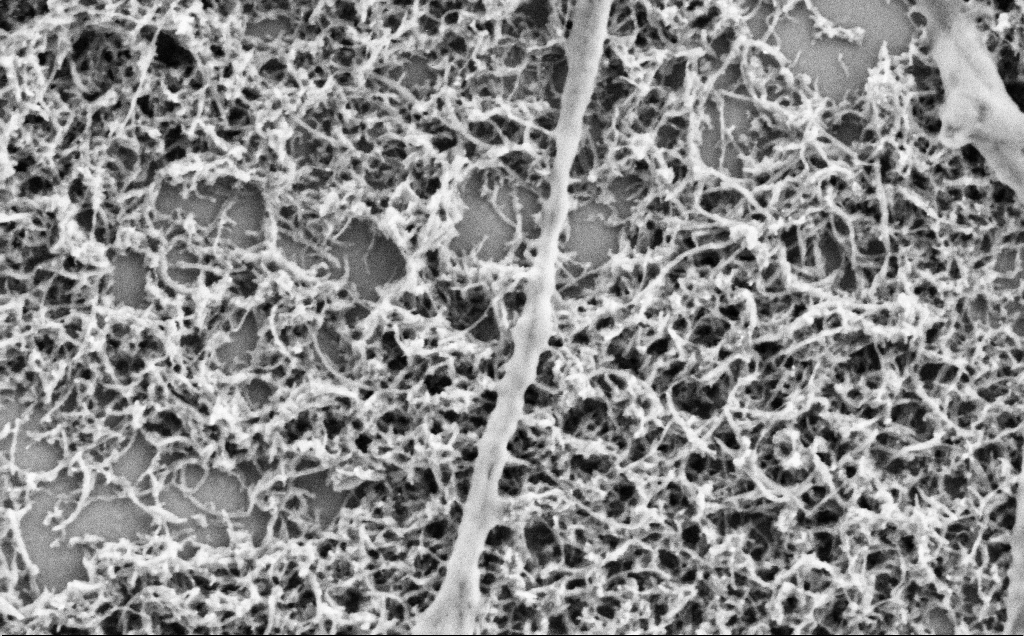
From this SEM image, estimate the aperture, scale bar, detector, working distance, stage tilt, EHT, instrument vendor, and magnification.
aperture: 30 µm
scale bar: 1000 nm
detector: SE2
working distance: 7.1 mm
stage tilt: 0°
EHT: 2 kV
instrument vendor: Zeiss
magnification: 50 K X